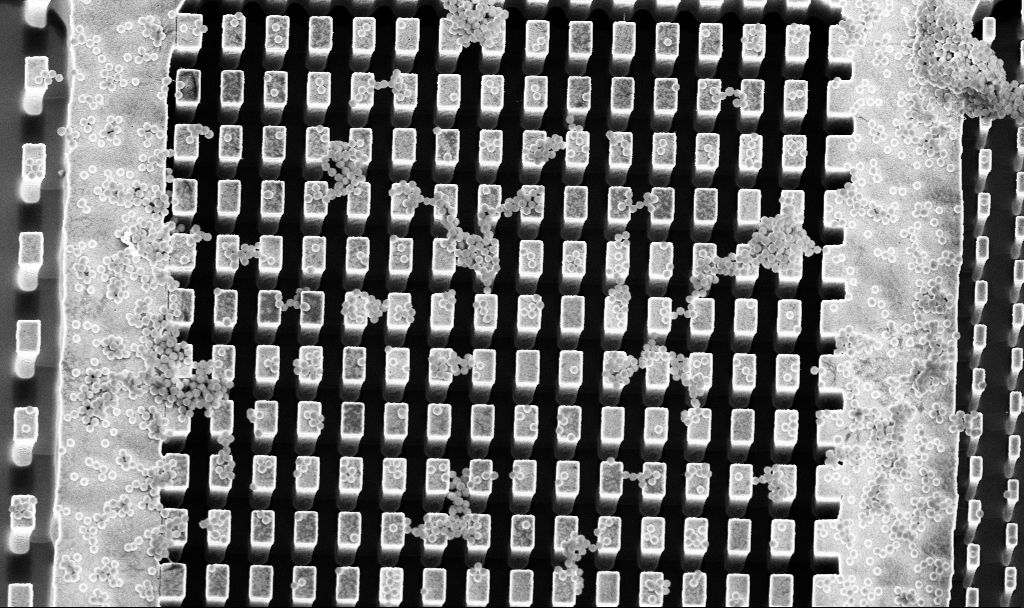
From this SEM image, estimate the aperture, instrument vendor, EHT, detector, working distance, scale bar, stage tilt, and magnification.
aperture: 30 µm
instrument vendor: Zeiss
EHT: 5 kV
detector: InLens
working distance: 3.3 mm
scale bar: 10000 nm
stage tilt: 8°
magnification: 5.06 K X